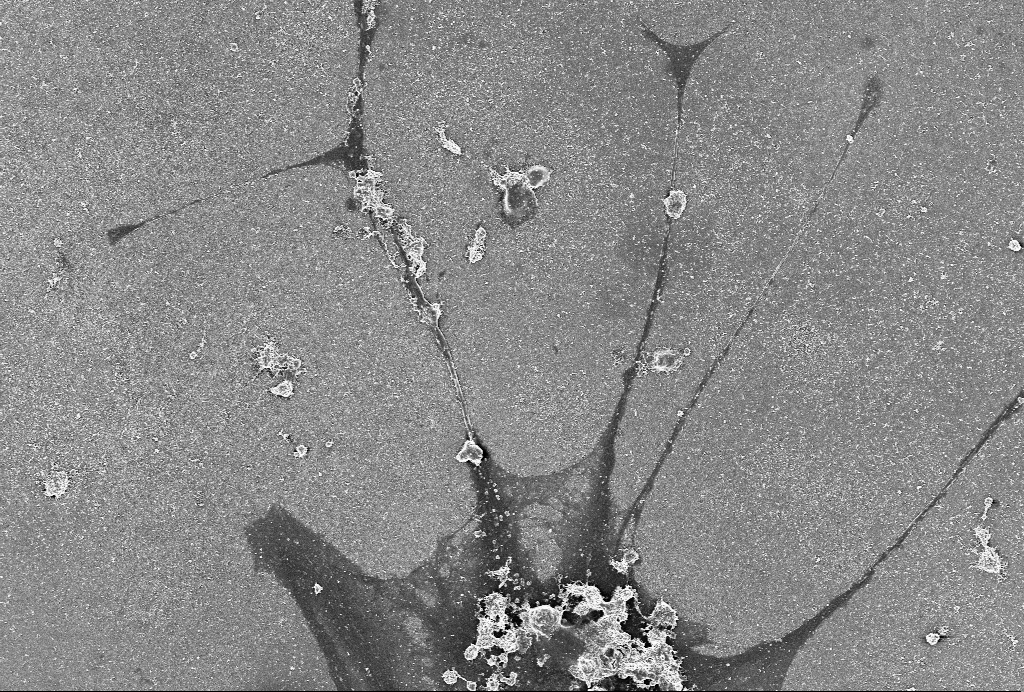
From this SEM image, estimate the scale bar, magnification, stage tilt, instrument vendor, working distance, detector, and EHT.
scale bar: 10000 nm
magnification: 1.5 K X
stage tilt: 0°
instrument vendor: Zeiss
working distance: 6 mm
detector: SE2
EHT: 4 kV